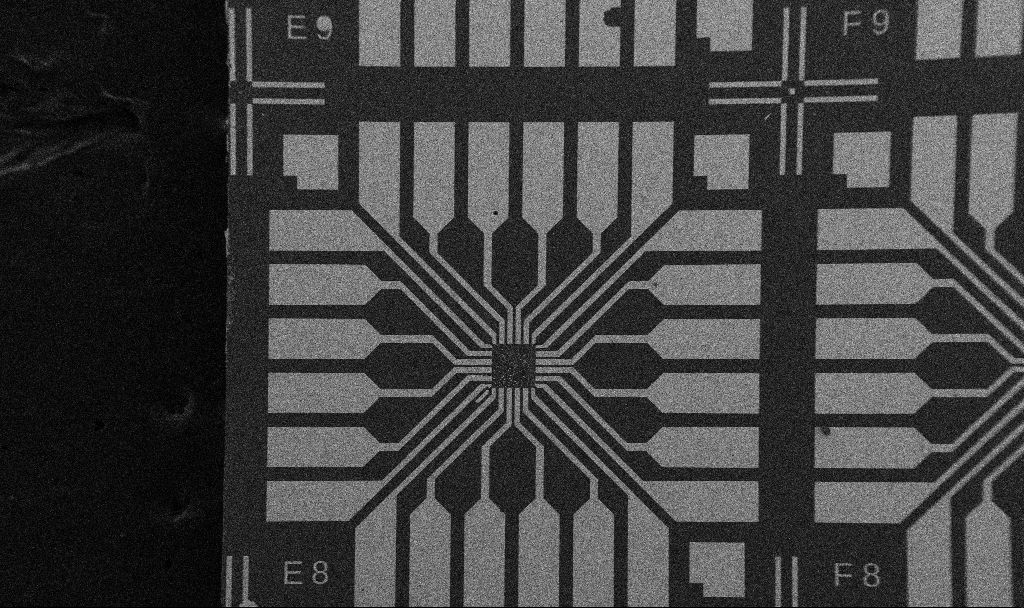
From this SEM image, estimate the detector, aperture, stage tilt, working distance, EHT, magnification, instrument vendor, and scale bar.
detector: SE2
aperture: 30 µm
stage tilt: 0°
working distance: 10.7 mm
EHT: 5 kV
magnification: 0.1 K X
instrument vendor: Zeiss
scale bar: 200000 nm